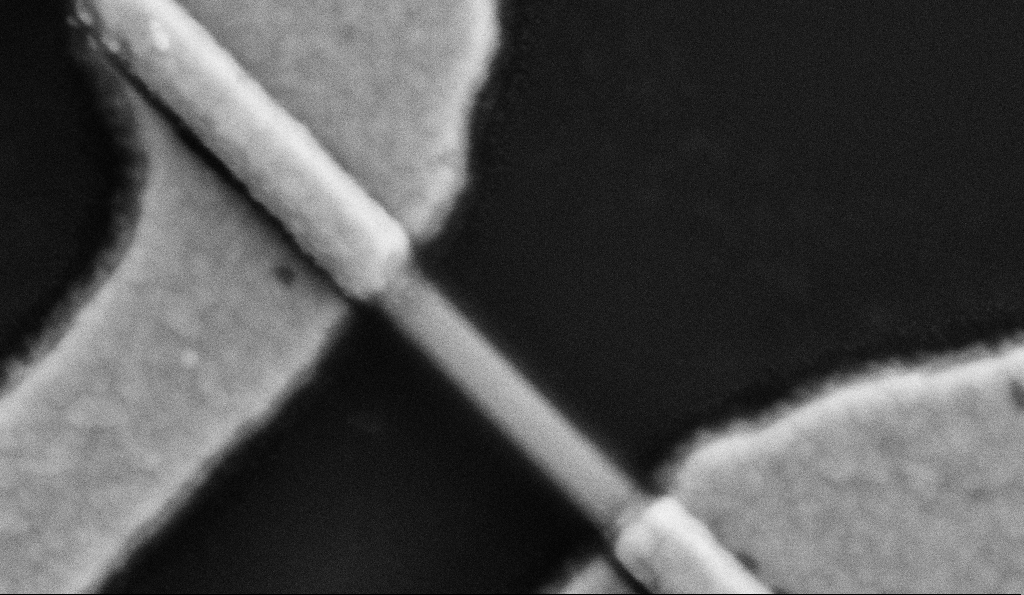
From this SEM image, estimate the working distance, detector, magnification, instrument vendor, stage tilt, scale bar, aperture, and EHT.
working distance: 9.6 mm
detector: SE2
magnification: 200 K X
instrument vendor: Zeiss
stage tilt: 0°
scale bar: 200 nm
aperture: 30 µm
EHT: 5 kV